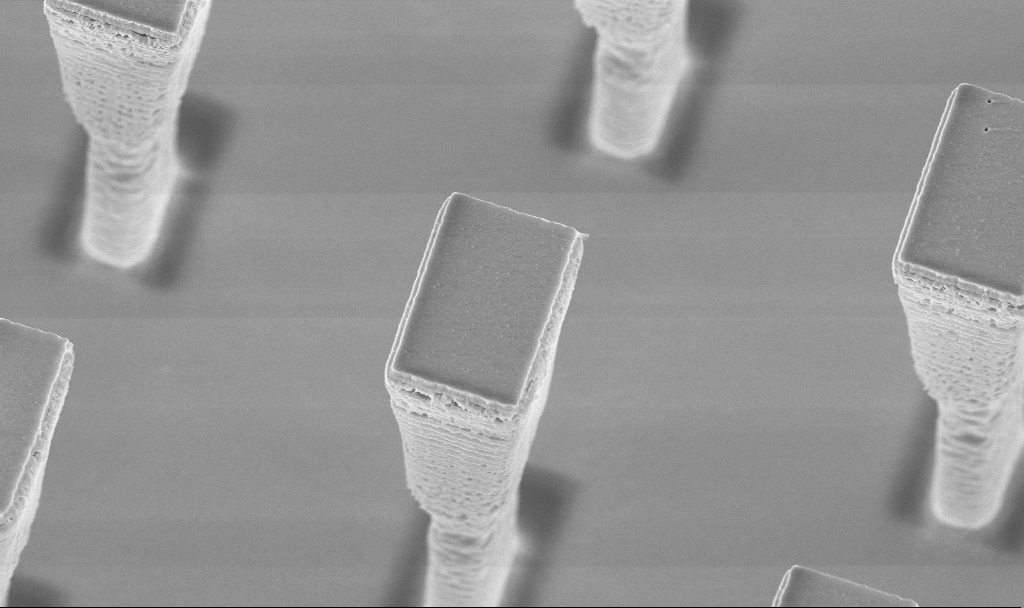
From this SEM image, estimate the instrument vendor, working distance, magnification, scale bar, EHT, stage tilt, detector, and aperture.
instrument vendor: Zeiss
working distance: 4.1 mm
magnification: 16.43 K X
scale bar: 2000 nm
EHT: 5 kV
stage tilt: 20°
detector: InLens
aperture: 30 µm